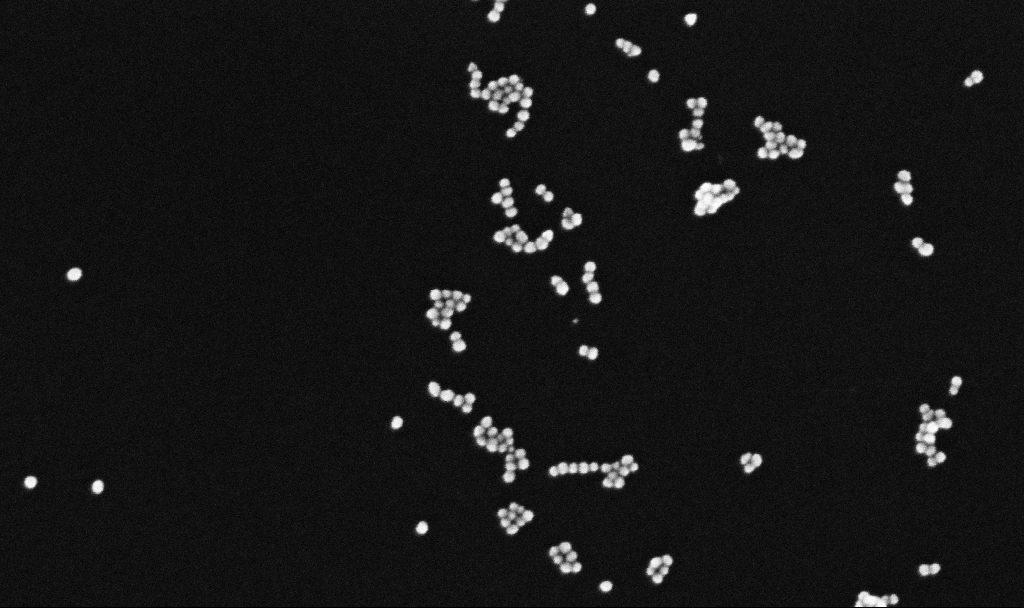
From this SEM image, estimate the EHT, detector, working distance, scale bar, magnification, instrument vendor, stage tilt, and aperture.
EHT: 10 kV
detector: InLens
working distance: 3.4 mm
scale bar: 100 nm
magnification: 300 K X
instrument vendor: Zeiss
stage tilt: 0°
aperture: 30 µm